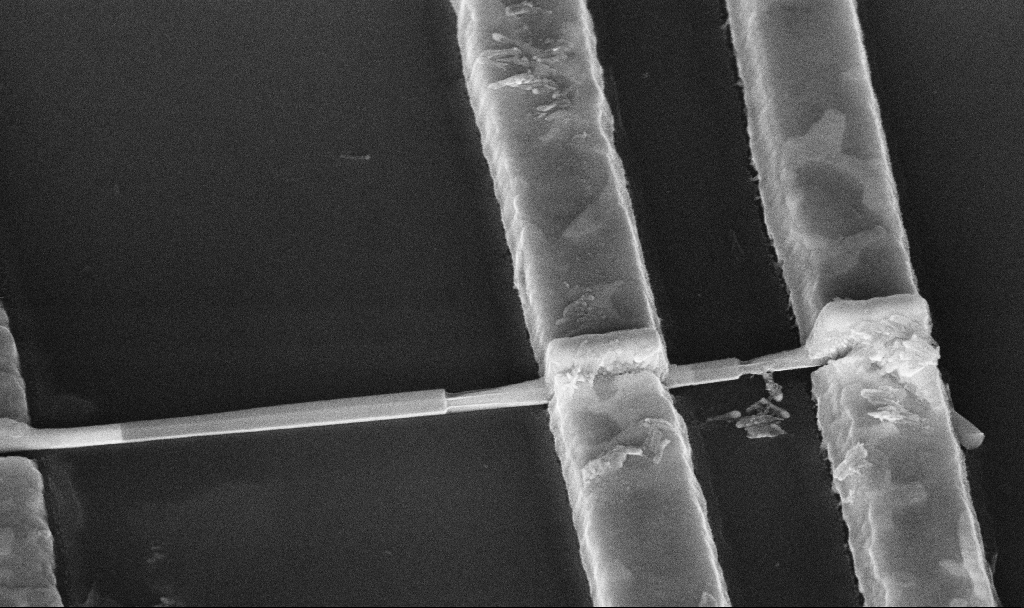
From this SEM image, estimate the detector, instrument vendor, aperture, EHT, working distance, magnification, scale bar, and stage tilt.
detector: InLens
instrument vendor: Zeiss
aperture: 30 µm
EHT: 10 kV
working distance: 8.6 mm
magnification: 77.34 K X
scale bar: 200 nm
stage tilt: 45°